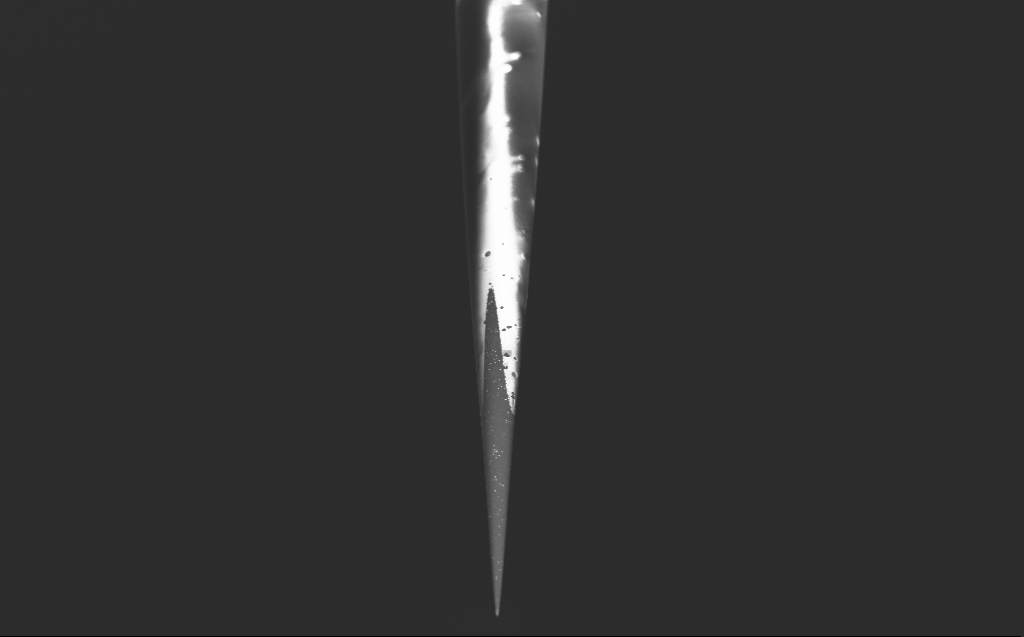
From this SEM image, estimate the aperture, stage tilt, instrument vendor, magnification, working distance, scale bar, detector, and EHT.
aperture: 30 µm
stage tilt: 45°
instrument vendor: Zeiss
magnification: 1.91 K X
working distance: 4 mm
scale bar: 10000 nm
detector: InLens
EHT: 5 kV